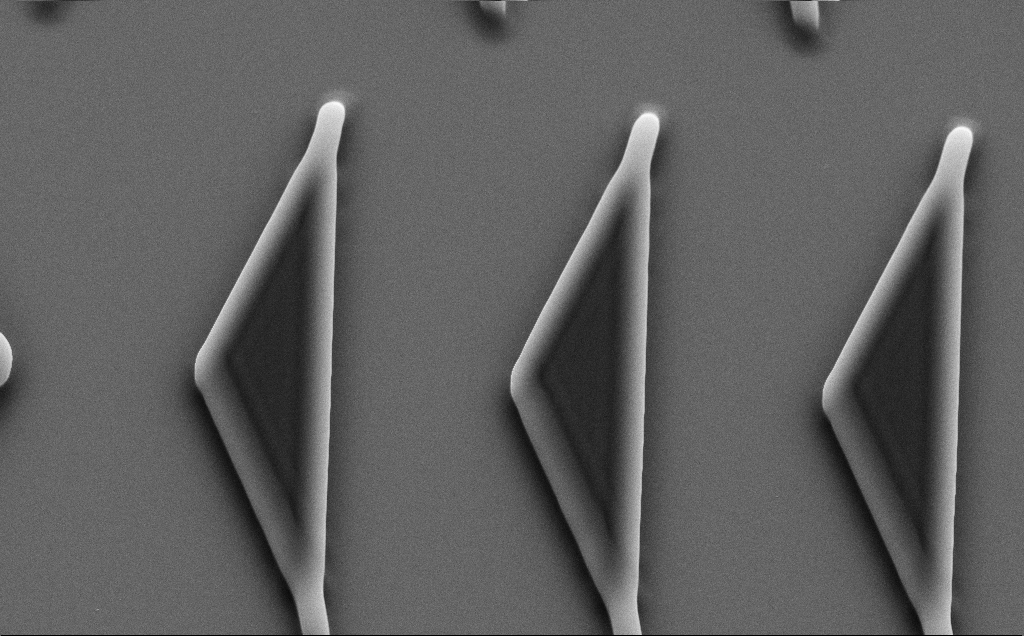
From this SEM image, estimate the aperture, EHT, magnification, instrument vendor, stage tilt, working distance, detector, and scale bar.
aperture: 30 µm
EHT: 10 kV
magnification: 6.81 K X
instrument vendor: Zeiss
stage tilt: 35°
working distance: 8 mm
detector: SE2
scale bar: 10000 nm